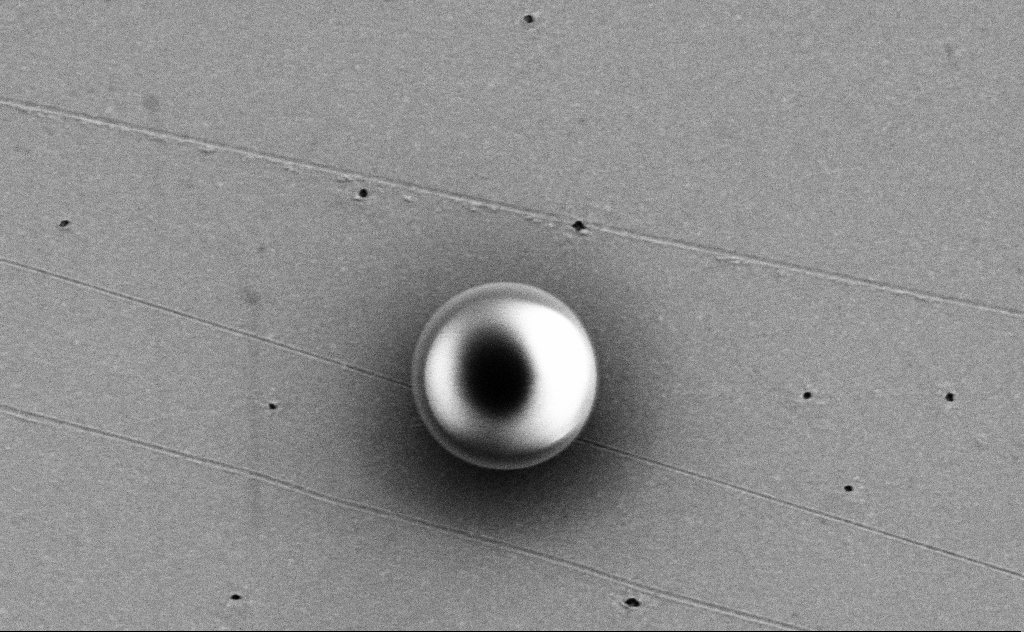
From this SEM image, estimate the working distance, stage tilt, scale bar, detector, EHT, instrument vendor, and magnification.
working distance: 10 mm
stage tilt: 0°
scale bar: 2000 nm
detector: SE2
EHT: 3 kV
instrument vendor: Zeiss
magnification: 26.65 K X